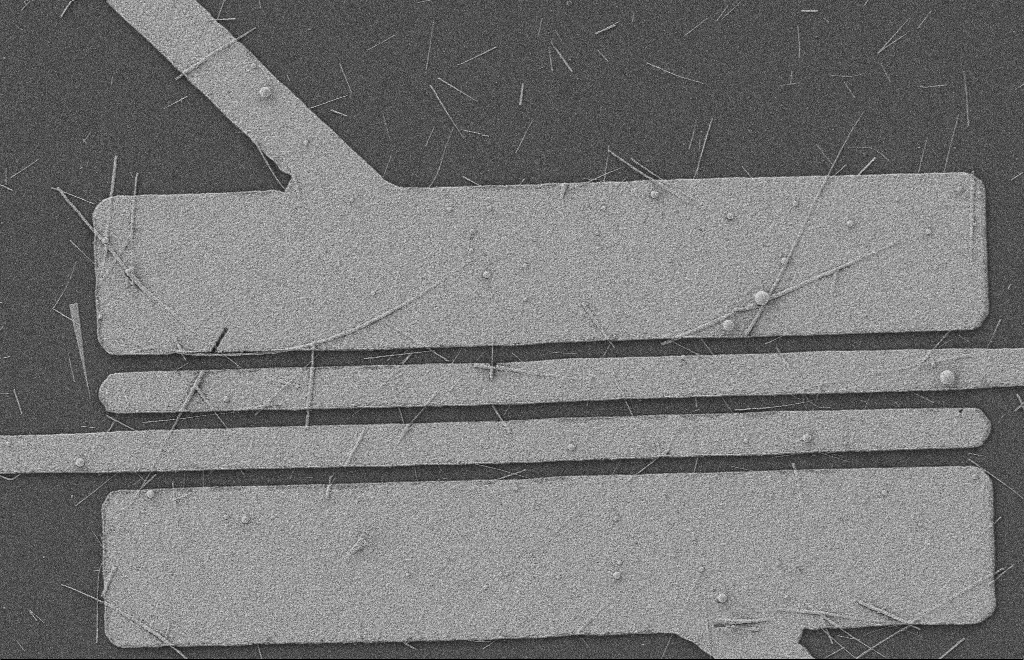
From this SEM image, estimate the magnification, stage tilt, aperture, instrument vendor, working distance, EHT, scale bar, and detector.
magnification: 5.37 K X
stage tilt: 0°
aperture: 20 µm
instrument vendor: Zeiss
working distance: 8 mm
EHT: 2 kV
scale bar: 2000 nm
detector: SE2